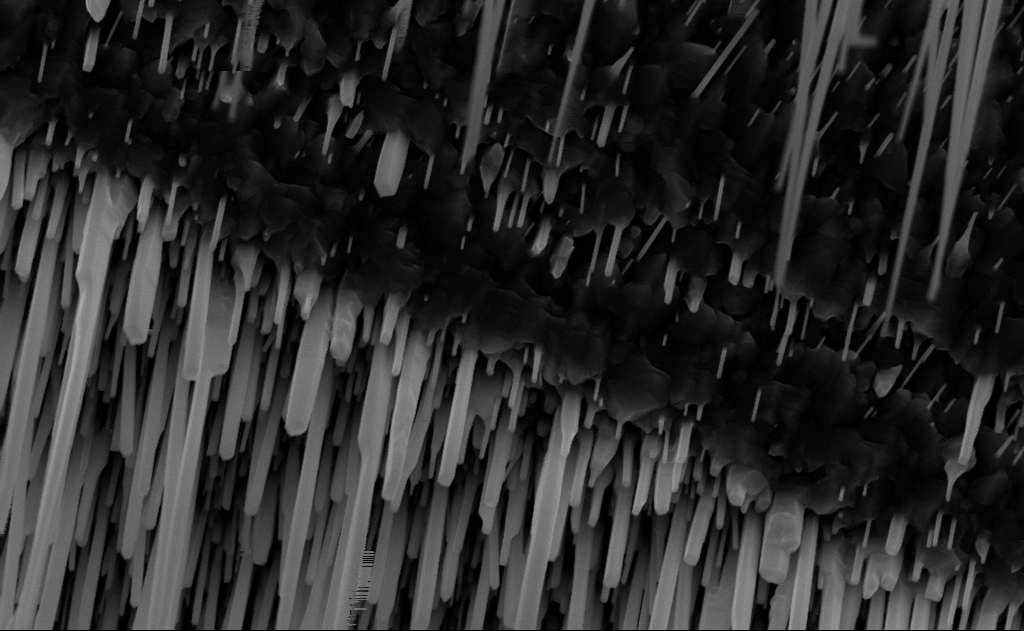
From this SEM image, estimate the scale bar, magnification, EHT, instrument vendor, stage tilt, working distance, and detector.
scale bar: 1000 nm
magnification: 40 K X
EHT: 10 kV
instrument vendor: Zeiss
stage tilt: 0°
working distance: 9 mm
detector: InLens